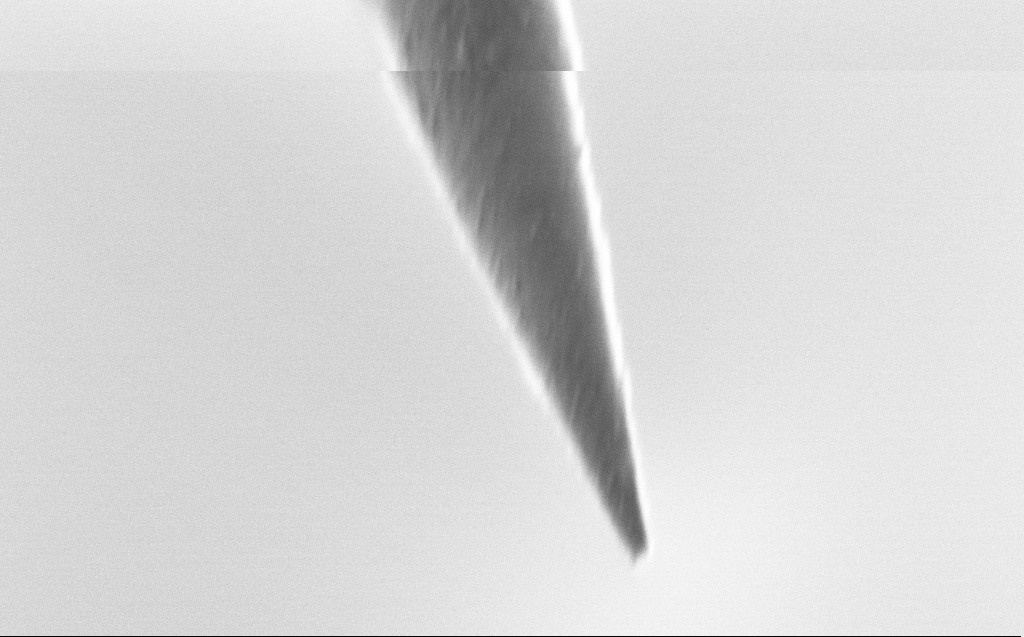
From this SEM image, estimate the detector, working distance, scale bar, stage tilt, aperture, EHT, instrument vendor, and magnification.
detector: SE2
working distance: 5 mm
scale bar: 200 nm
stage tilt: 39.3°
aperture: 30 µm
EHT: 0.8 kV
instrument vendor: Zeiss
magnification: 96.26 K X